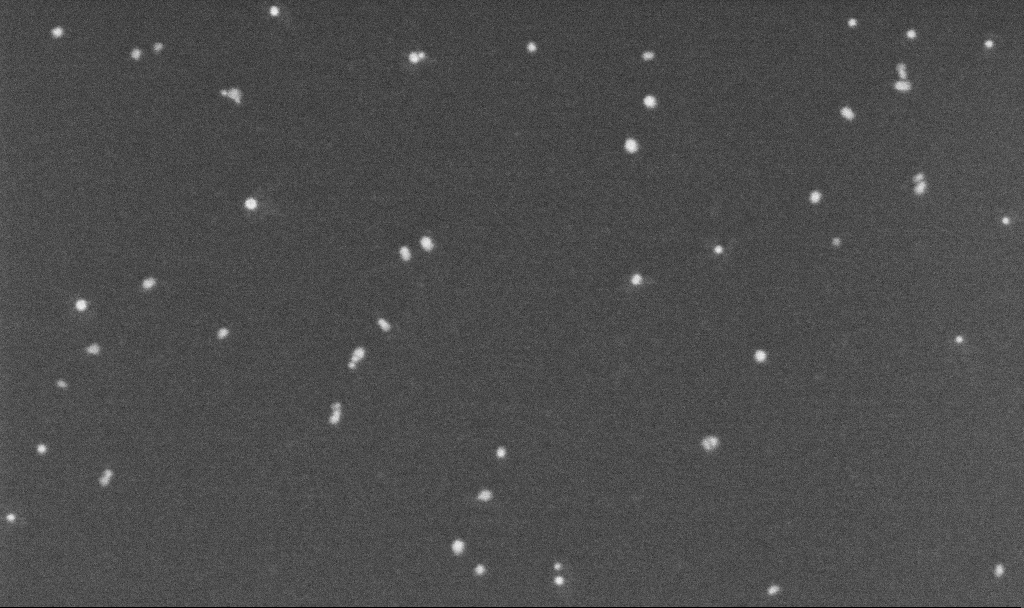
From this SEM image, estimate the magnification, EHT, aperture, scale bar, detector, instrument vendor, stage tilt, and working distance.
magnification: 250 K X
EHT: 5 kV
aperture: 30 µm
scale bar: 100 nm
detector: InLens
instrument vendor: Zeiss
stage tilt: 0°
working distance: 3.2 mm